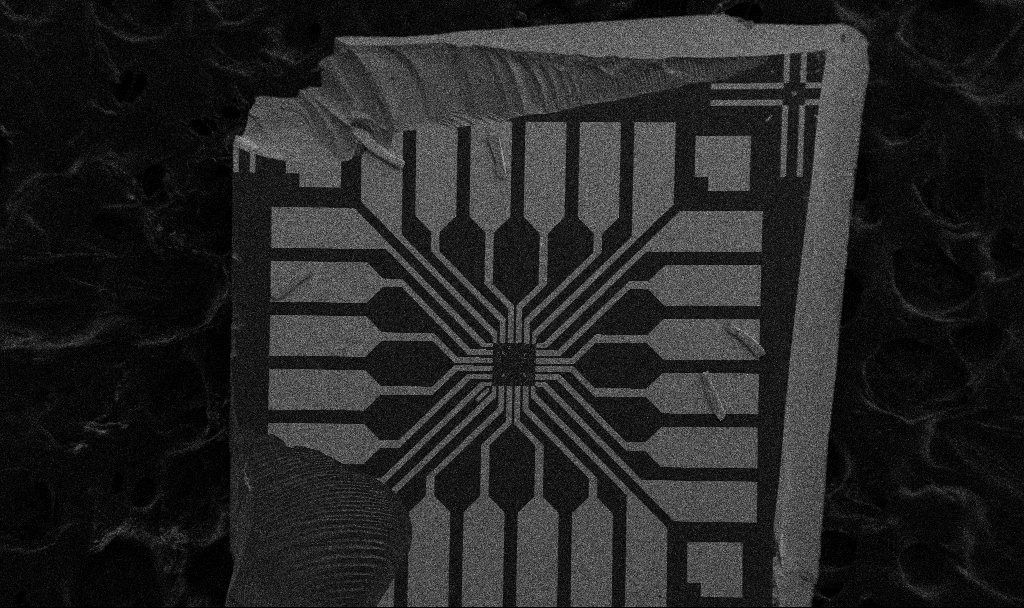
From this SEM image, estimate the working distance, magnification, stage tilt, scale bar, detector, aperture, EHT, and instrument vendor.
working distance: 8.7 mm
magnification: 0.1 K X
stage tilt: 0°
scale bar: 200000 nm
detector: SE2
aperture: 30 µm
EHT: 5 kV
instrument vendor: Zeiss